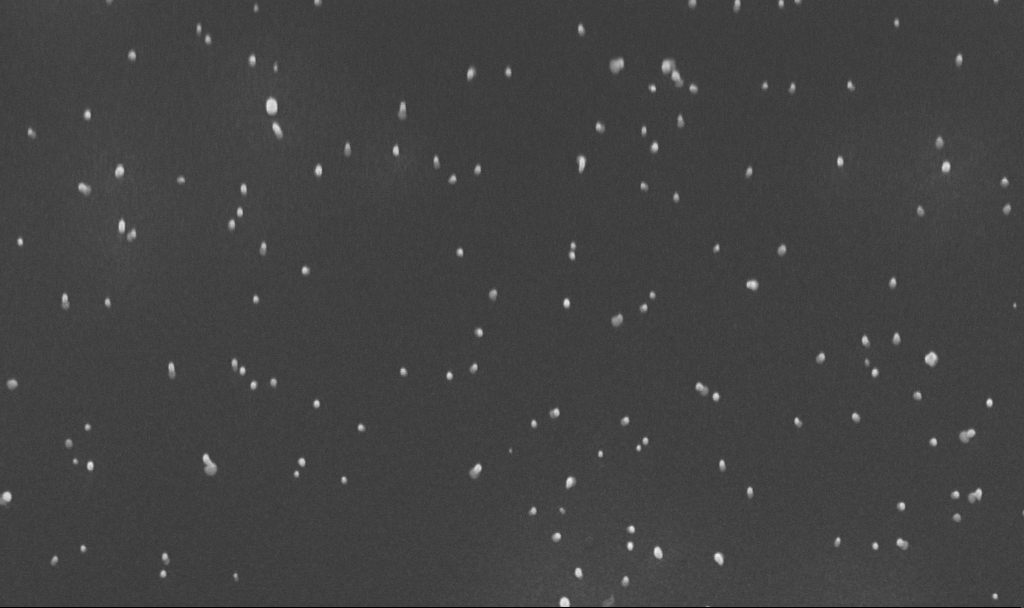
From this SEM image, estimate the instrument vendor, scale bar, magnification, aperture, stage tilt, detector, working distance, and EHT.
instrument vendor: Zeiss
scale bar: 200 nm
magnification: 100 K X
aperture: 30 µm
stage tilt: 45°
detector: InLens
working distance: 4.9 mm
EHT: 10 kV